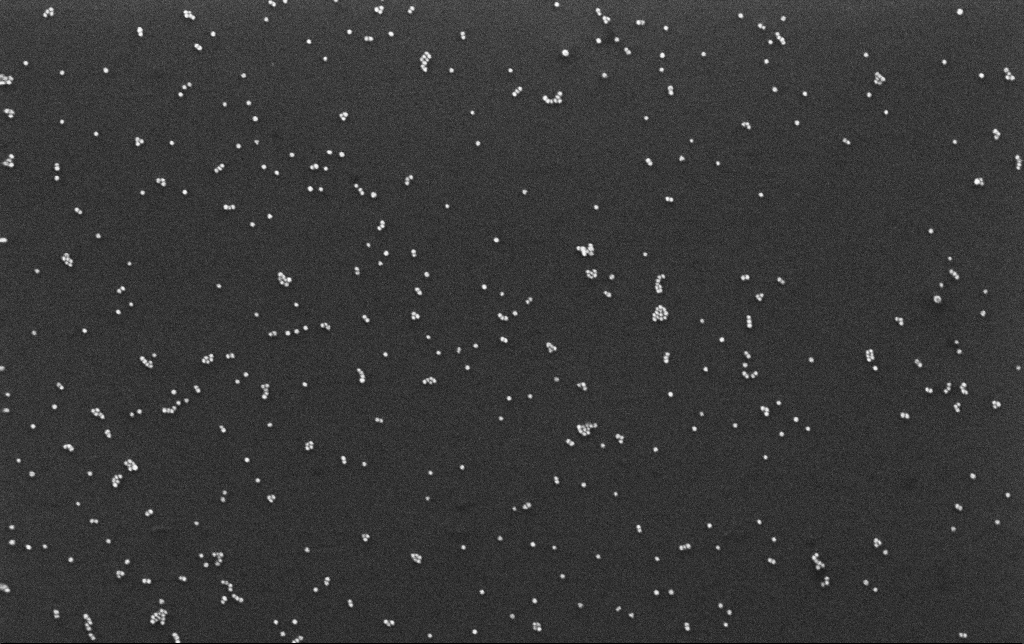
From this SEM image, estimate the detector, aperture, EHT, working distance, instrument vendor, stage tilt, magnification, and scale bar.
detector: InLens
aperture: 30 µm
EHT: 10 kV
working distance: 3 mm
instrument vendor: Zeiss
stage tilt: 0°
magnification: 100 K X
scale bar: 200 nm